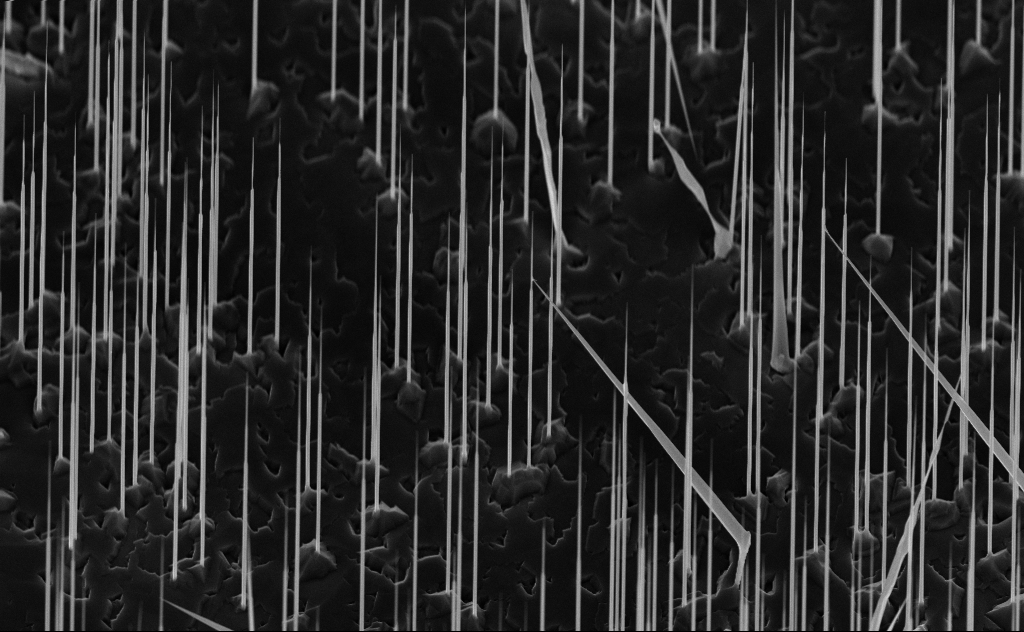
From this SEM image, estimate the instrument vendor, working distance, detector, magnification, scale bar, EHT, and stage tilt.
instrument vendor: Zeiss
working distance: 6 mm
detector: InLens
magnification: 10 K X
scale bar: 2000 nm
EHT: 10 kV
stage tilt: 45°